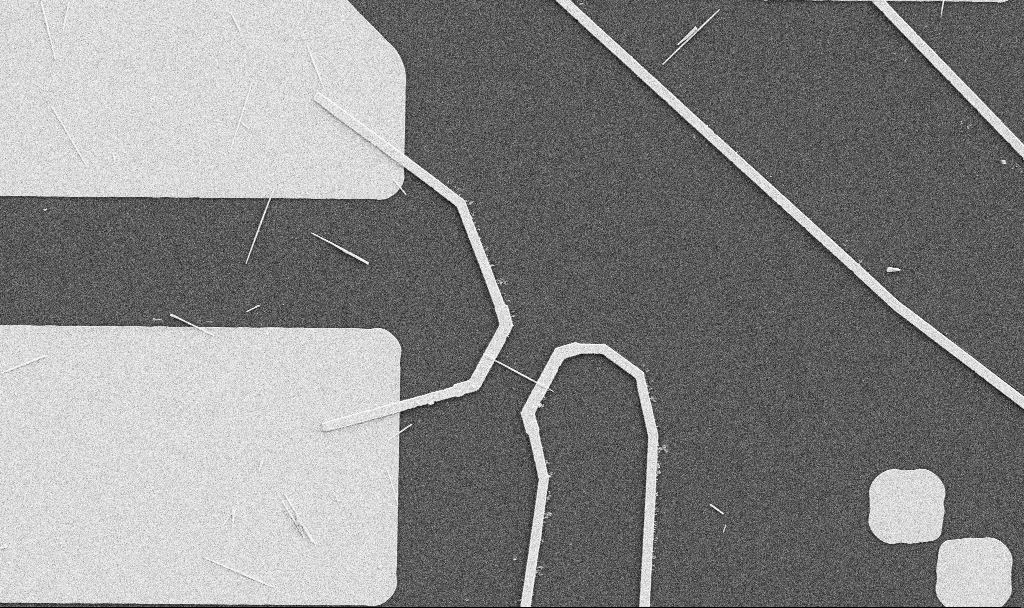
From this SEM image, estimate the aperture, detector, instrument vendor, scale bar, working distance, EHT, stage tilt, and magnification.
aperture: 30 µm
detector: SE2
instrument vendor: Zeiss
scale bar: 10000 nm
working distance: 10.7 mm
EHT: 5 kV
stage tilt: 0°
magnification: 5 K X